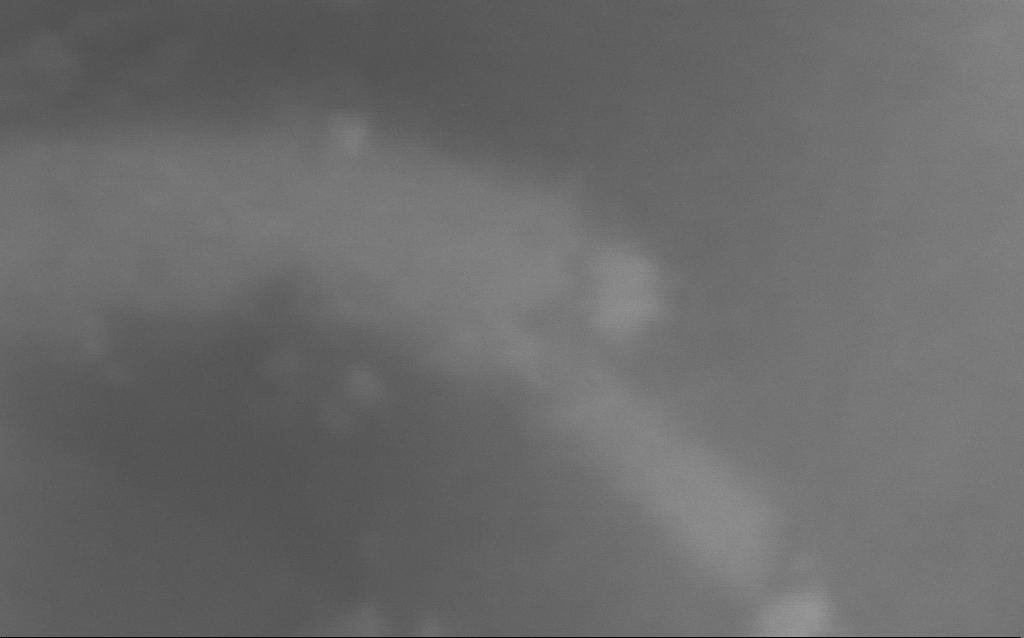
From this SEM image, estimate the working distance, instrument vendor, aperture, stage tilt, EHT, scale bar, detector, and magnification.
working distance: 4 mm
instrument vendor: Zeiss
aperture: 30 µm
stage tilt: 0°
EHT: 5 kV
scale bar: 20 nm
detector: InLens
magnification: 1290.63 K X